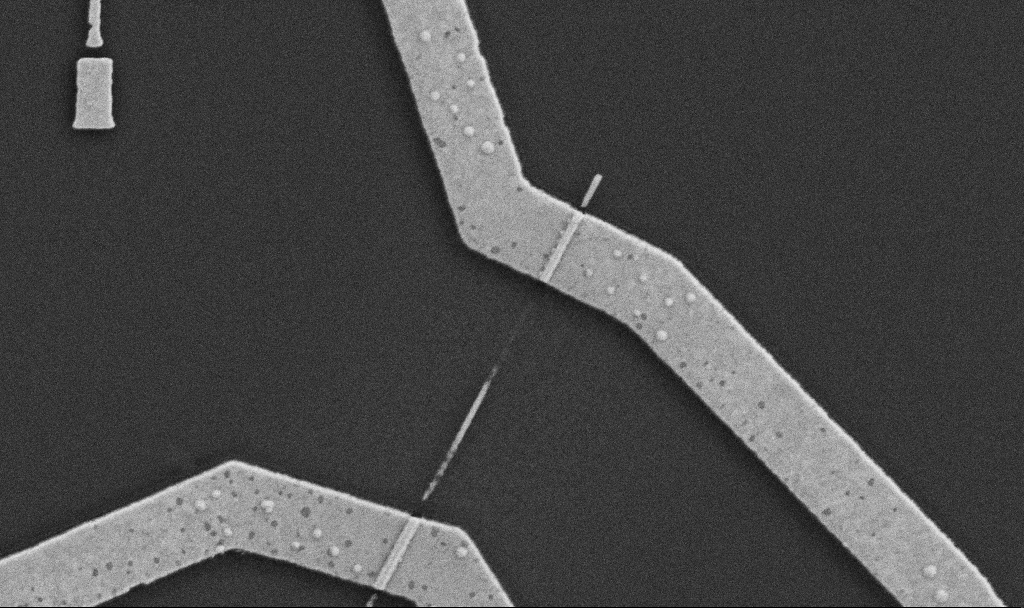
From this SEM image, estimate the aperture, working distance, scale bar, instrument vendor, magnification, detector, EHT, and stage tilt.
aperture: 30 µm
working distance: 7.6 mm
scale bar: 1000 nm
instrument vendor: Zeiss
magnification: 30 K X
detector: SE2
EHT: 5 kV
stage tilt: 0°